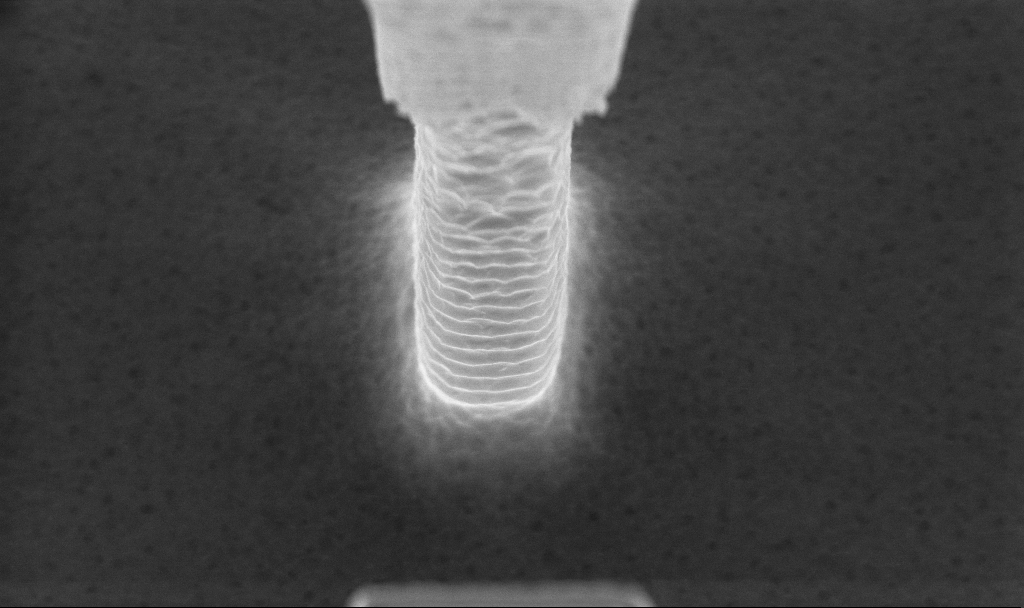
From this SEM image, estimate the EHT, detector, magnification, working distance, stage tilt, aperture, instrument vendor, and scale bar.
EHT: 5 kV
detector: InLens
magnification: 34.73 K X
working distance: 4.3 mm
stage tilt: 20°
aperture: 30 µm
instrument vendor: Zeiss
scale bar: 1000 nm